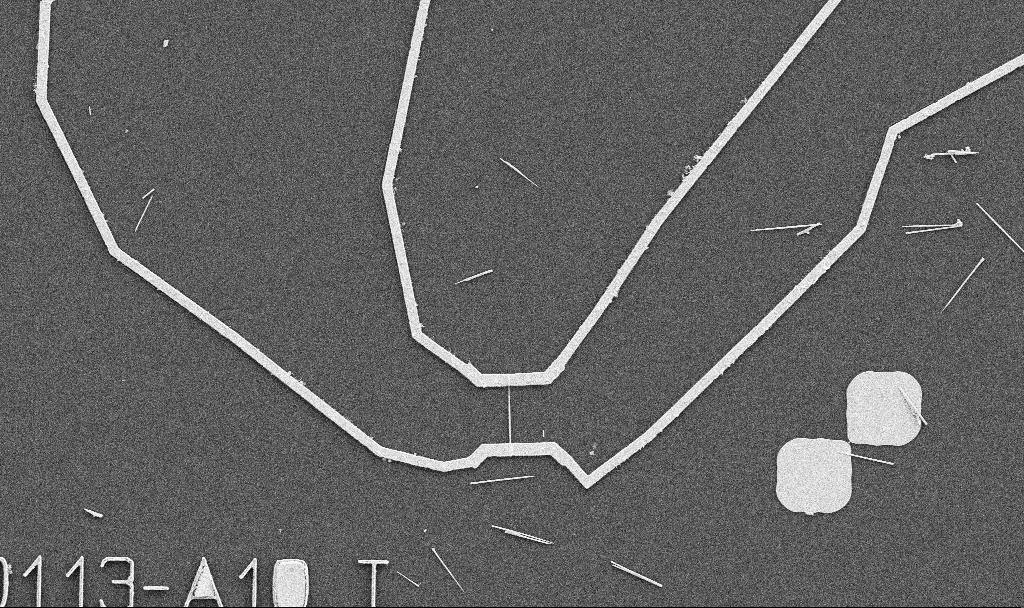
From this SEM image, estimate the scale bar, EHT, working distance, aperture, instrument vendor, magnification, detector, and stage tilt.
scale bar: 10000 nm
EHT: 5 kV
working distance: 10.7 mm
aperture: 30 µm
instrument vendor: Zeiss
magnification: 5 K X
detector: SE2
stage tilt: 0°